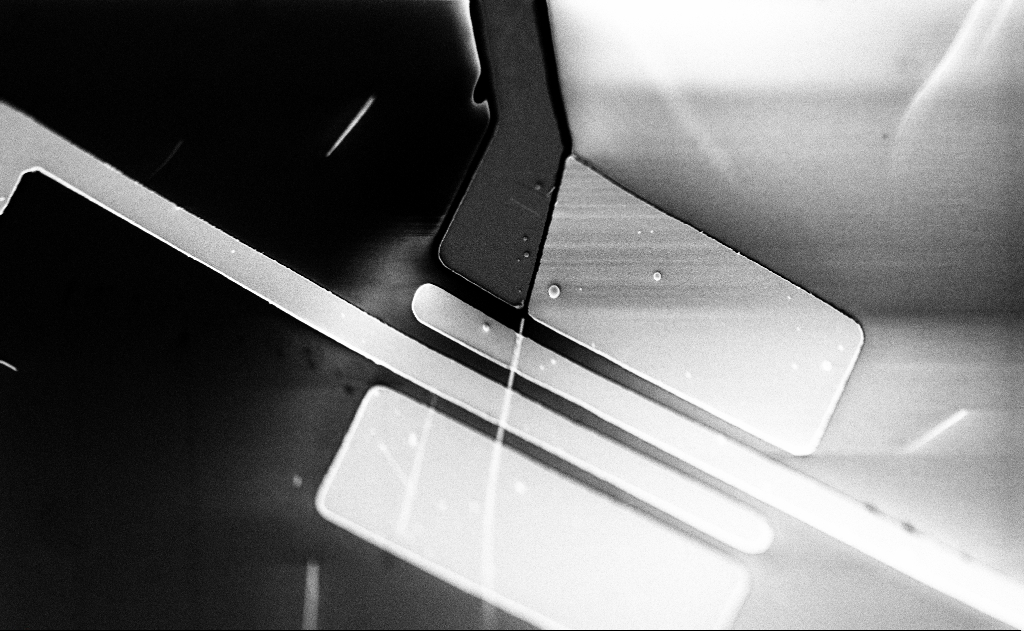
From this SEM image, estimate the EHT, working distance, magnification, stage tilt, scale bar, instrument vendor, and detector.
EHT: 5 kV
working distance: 20 mm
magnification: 4.98 K X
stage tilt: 0°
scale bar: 10000 nm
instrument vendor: Zeiss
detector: SE2